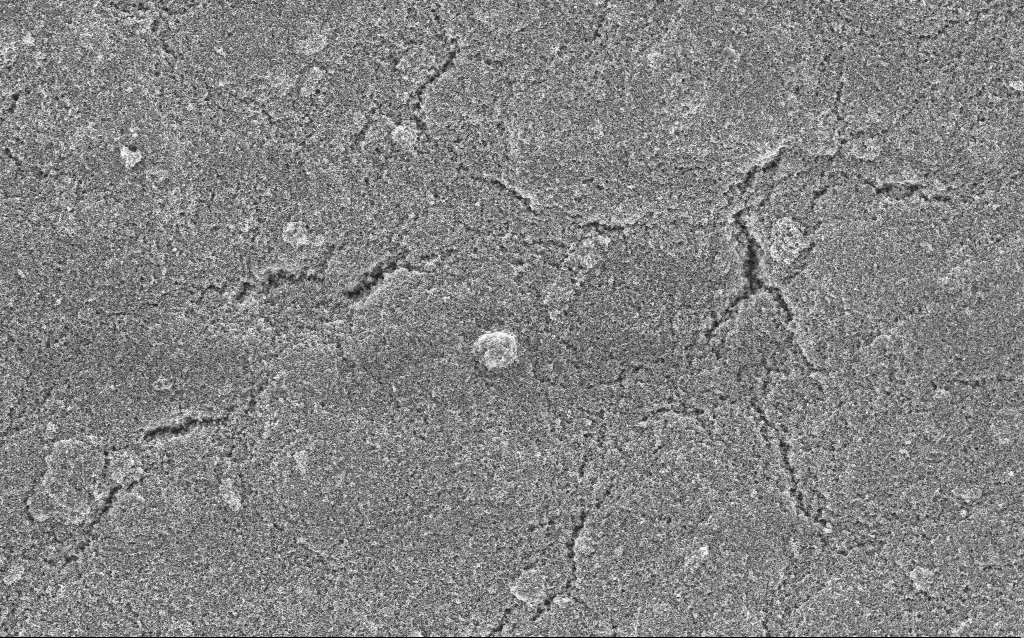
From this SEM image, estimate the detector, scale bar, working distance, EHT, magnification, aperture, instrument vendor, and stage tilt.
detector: InLens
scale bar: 10000 nm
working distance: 4.4 mm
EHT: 5 kV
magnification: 6.61 K X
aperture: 30 µm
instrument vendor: Zeiss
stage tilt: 0°